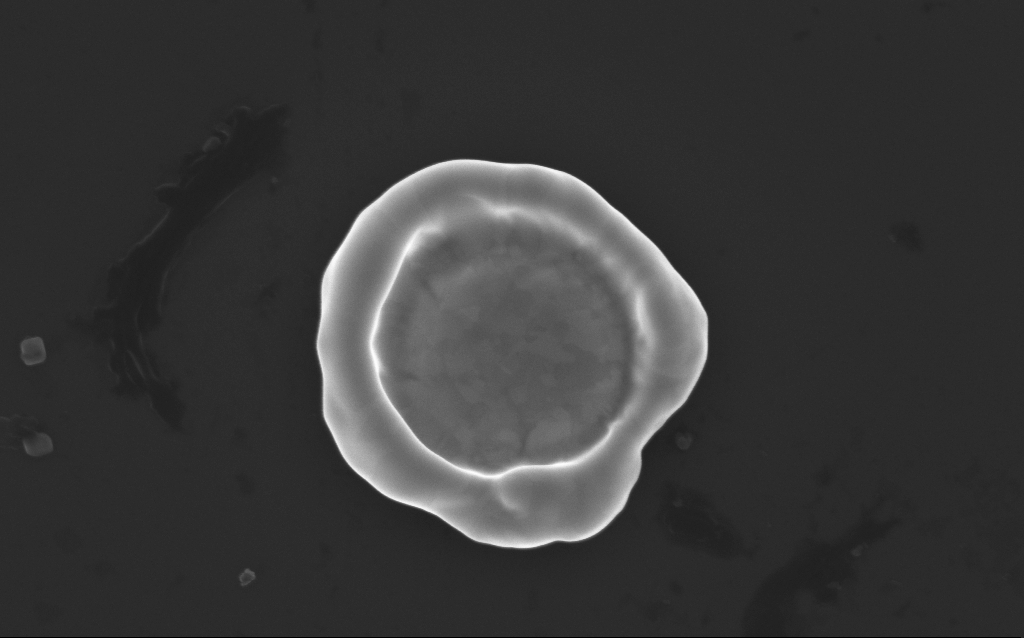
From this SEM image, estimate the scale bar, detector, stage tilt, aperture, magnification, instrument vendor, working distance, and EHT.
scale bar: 1000 nm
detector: InLens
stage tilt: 0°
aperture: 30 µm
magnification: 51.5 K X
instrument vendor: Zeiss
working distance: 4 mm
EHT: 10 kV